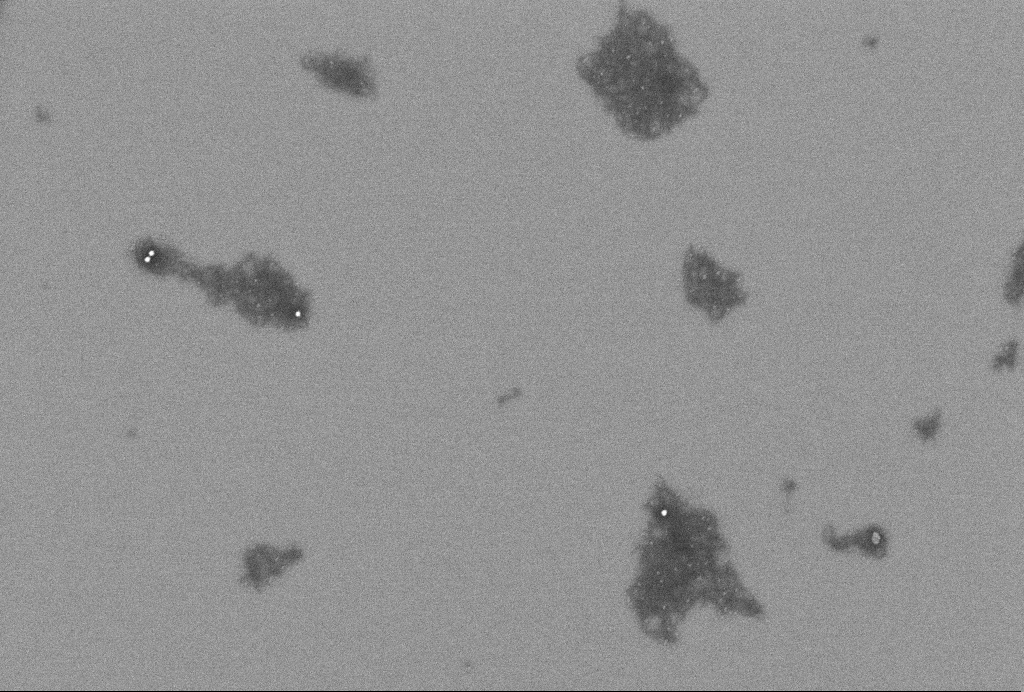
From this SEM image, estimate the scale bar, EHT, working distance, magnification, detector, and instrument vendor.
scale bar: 200 nm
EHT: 2 kV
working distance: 3.3 mm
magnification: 64.42 K X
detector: InLens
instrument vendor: Zeiss